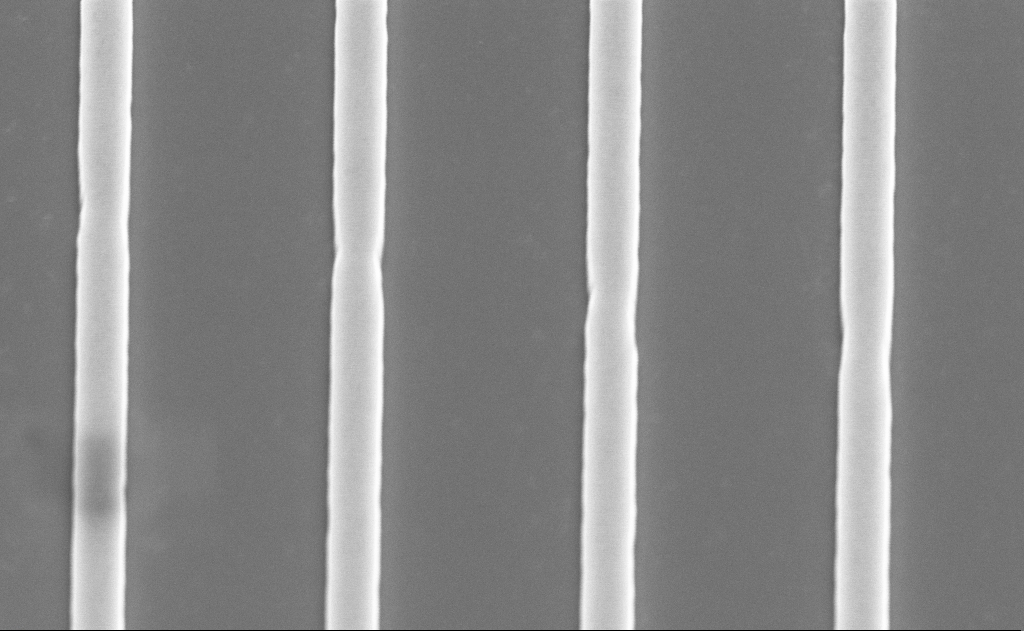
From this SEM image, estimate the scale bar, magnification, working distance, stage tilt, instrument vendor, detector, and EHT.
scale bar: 1000 nm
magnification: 23.2 K X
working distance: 13 mm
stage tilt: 55°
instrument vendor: Zeiss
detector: SE2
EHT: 5 kV